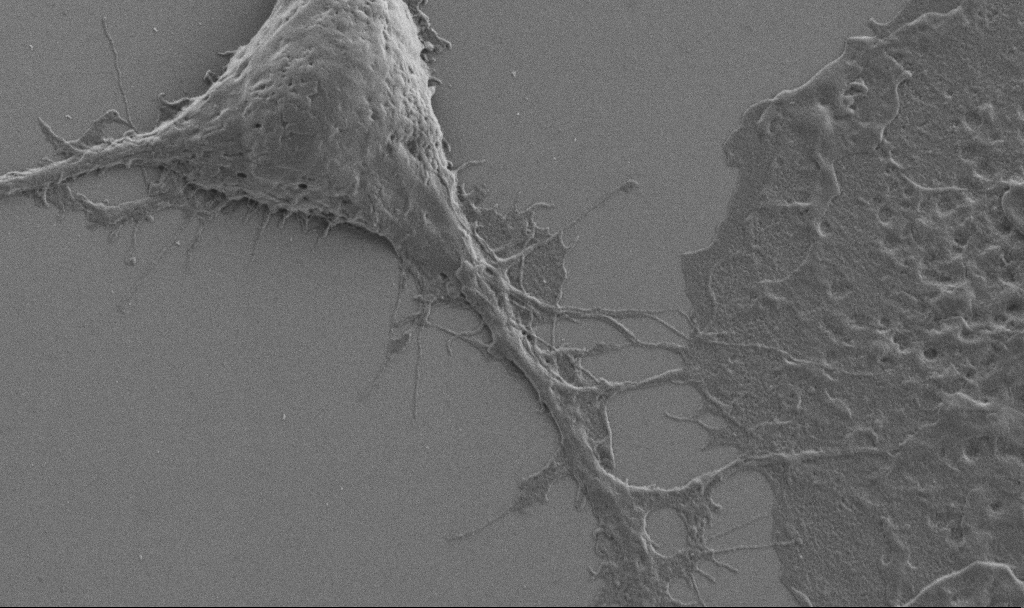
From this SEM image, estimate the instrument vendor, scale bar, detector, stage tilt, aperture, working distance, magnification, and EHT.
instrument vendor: Zeiss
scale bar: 2000 nm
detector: SE2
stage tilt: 0°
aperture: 30 µm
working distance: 6.9 mm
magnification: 10 K X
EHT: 1 kV